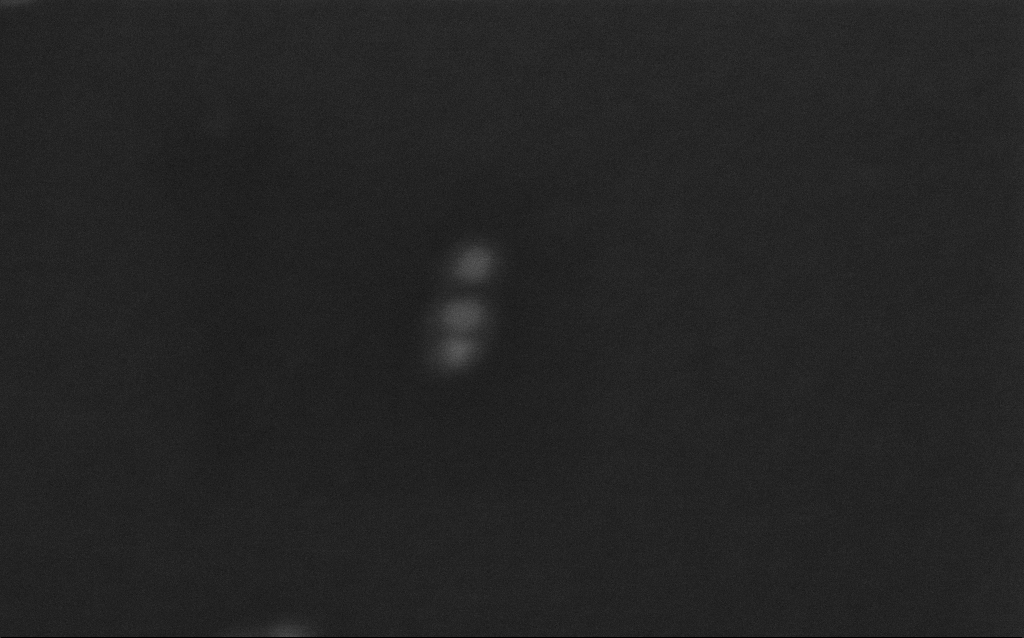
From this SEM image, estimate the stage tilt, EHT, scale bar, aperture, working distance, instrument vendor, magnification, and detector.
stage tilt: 0°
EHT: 10 kV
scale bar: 20 nm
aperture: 30 µm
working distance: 7 mm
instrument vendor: Zeiss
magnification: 1052.98 K X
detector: InLens